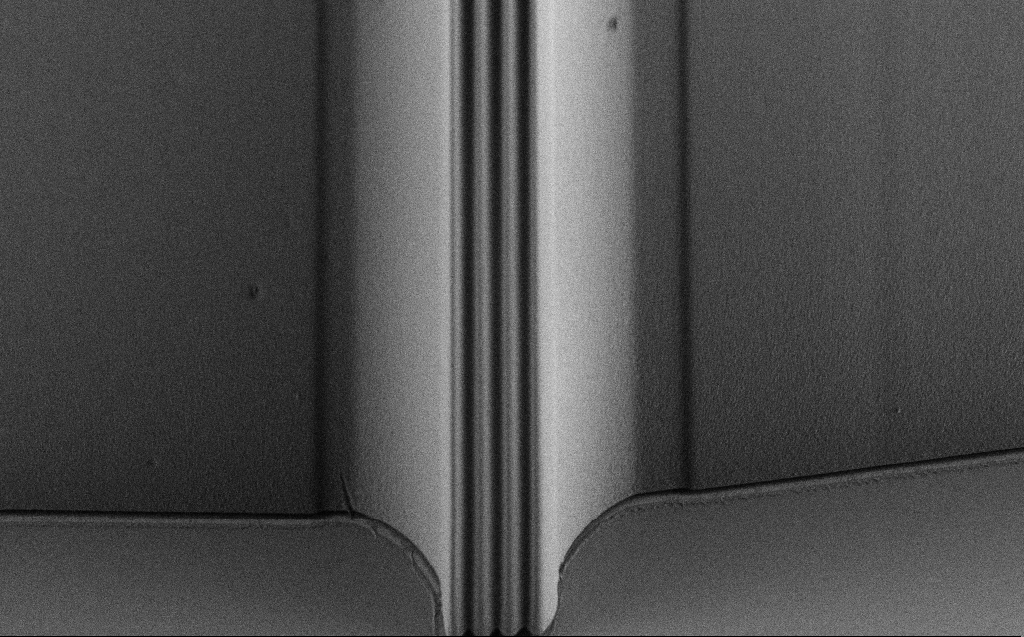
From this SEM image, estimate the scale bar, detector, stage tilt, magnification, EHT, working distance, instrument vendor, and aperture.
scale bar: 20000 nm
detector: SE2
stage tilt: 45°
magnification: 0.939 K X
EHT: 0.9 kV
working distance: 4 mm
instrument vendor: Zeiss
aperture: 30 µm